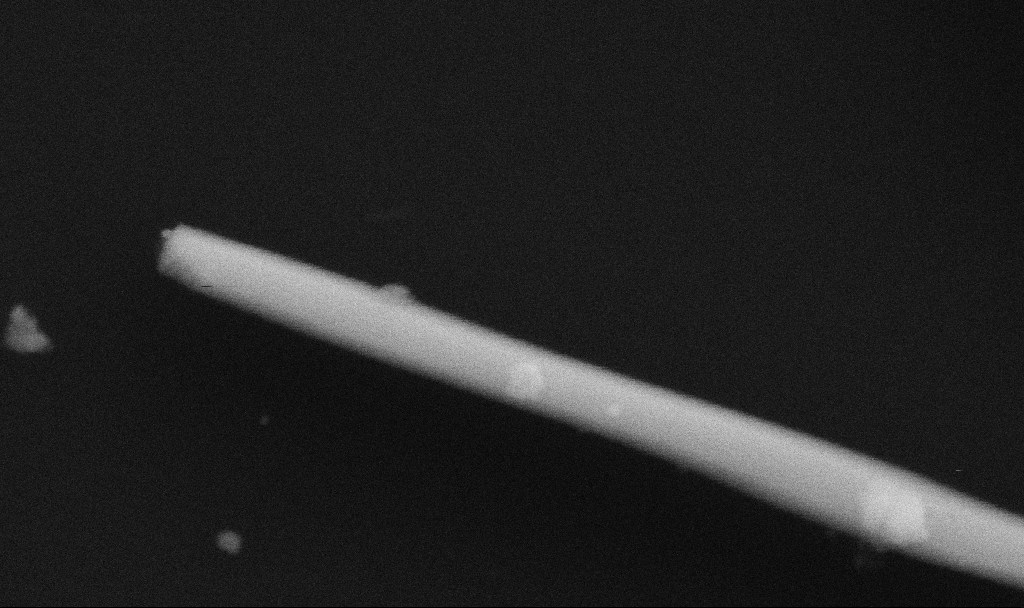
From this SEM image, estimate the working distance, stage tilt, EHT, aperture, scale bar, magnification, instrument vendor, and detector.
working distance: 6.7 mm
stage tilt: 0°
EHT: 5 kV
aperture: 30 µm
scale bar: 200 nm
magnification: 290.4 K X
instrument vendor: Zeiss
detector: SE2